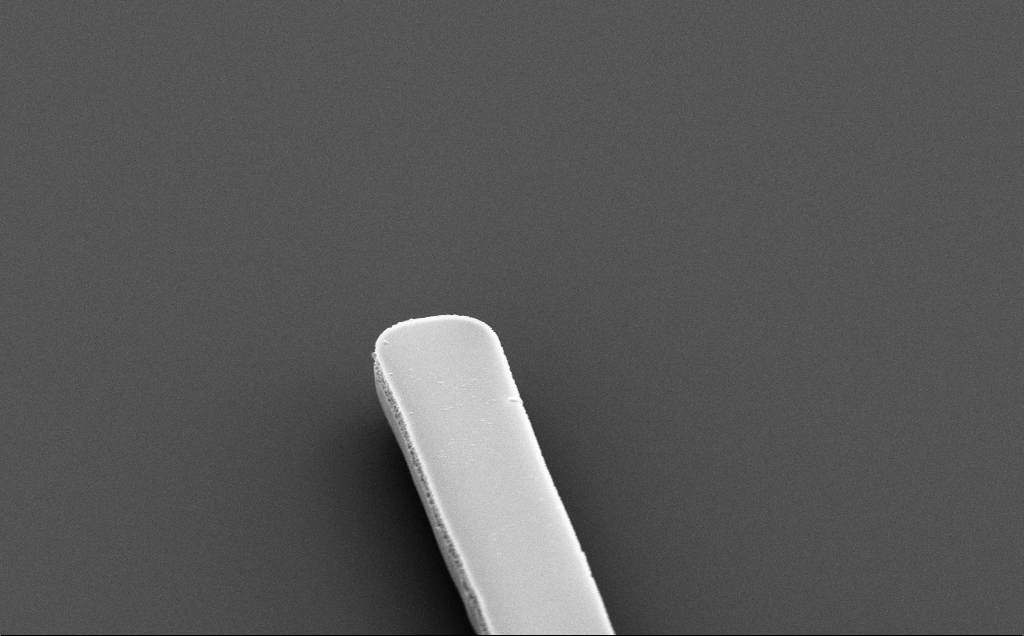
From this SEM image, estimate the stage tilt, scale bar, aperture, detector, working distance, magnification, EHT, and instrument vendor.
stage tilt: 50°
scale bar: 2000 nm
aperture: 30 µm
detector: SE2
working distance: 10 mm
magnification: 9.61 K X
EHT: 5 kV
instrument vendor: Zeiss